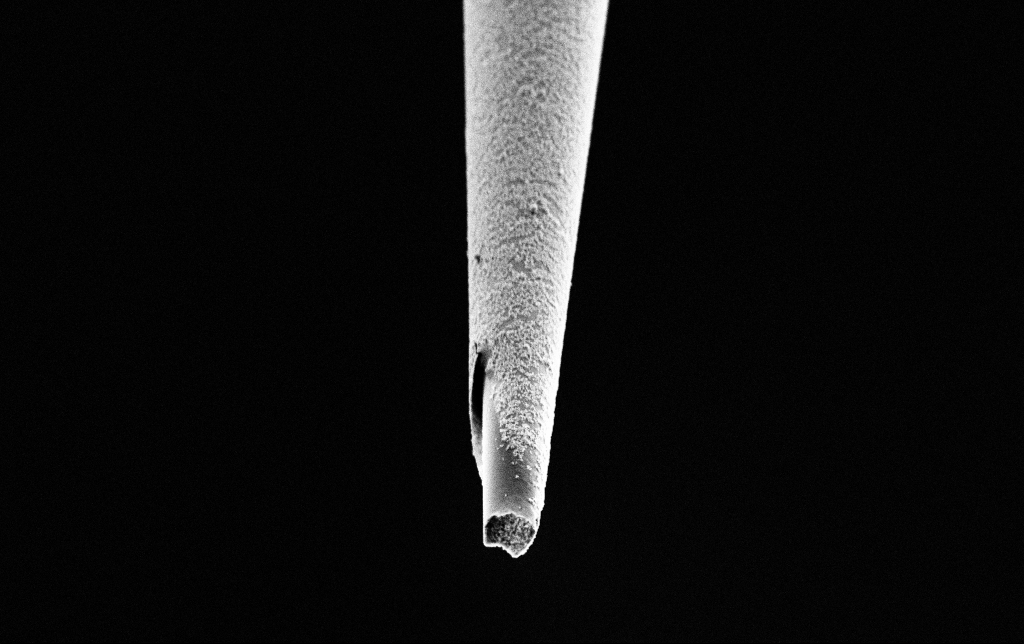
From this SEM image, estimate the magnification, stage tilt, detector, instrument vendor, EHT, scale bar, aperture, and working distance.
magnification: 15 K X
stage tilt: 45°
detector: SE2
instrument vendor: Zeiss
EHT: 2 kV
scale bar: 1000 nm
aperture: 30 µm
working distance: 7.3 mm